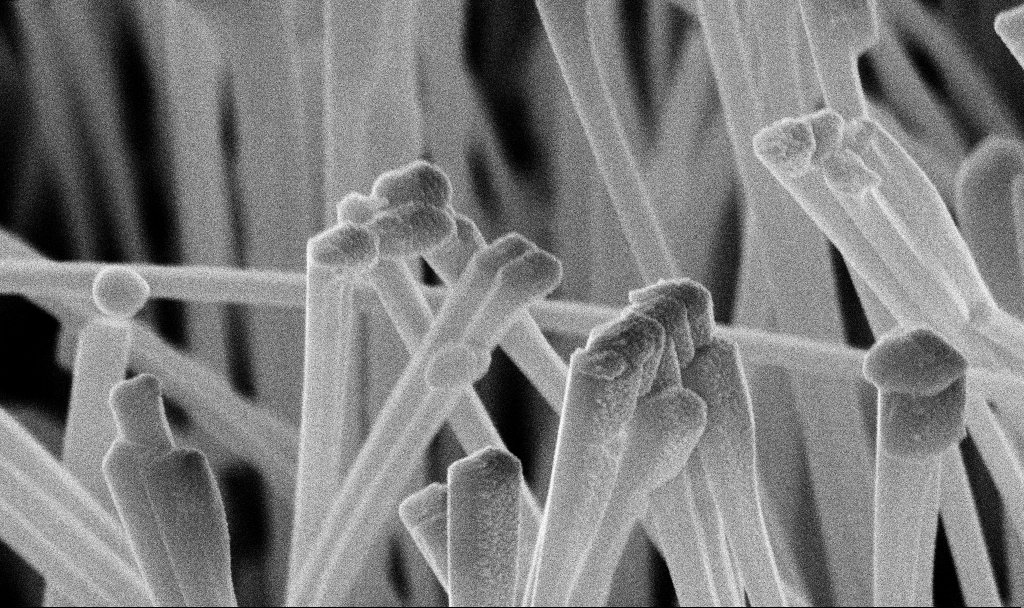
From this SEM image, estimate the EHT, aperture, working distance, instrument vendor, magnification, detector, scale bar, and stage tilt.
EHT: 10 kV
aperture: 30 µm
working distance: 7.2 mm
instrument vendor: Zeiss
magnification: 113.29 K X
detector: InLens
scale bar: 200 nm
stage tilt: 45°